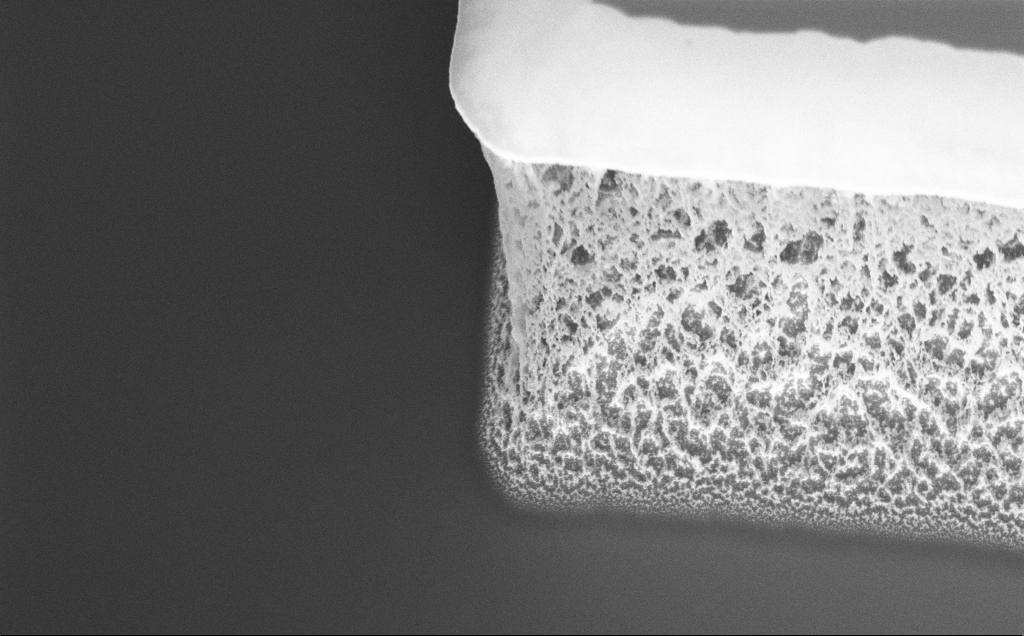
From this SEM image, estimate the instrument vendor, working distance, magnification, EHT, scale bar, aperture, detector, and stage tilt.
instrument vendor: Zeiss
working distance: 8 mm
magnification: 10.7 K X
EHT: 5 kV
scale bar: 2000 nm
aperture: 30 µm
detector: InLens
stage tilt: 45°